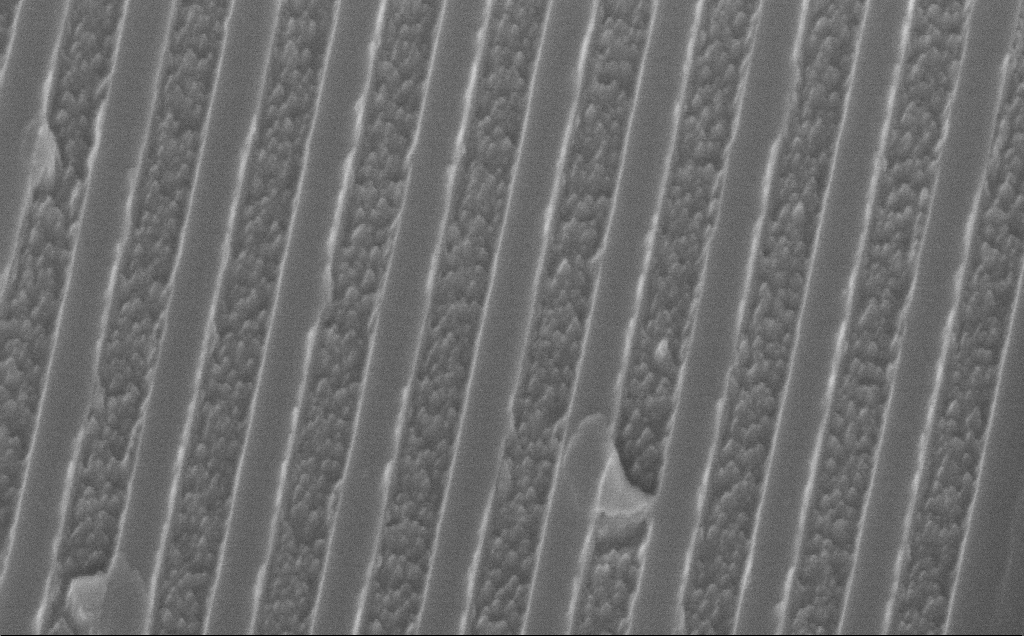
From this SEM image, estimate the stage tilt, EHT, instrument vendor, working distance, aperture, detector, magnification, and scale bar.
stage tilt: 38°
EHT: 10 kV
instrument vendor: Zeiss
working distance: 6 mm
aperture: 30 µm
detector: InLens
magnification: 75.37 K X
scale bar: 200 nm